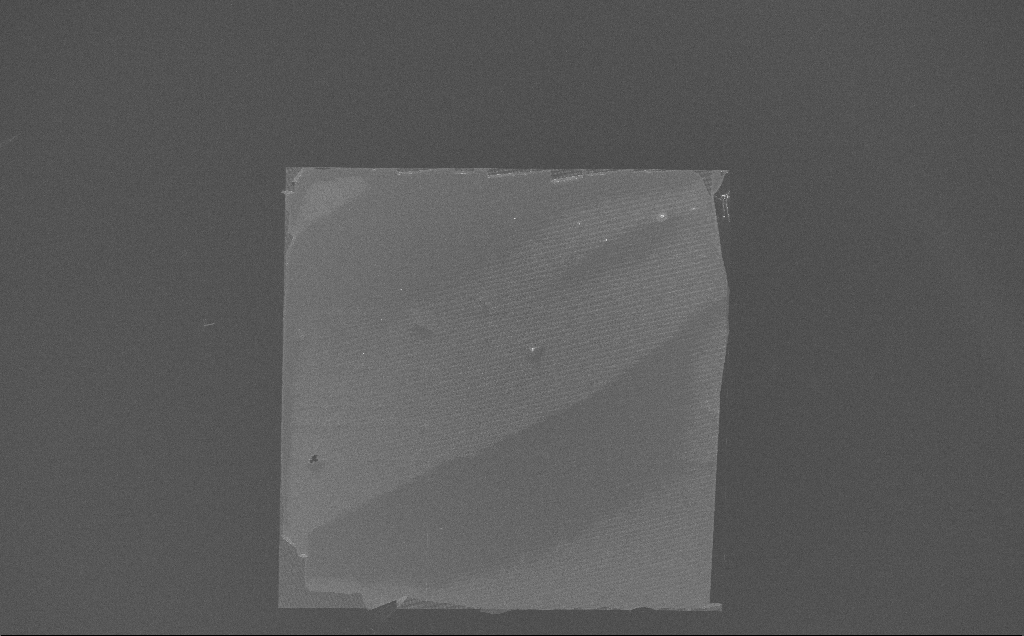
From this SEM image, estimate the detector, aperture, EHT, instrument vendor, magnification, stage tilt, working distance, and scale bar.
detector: InLens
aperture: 30 µm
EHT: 10 kV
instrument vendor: Zeiss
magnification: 0.219 K X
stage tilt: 0°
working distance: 7 mm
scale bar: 100000 nm